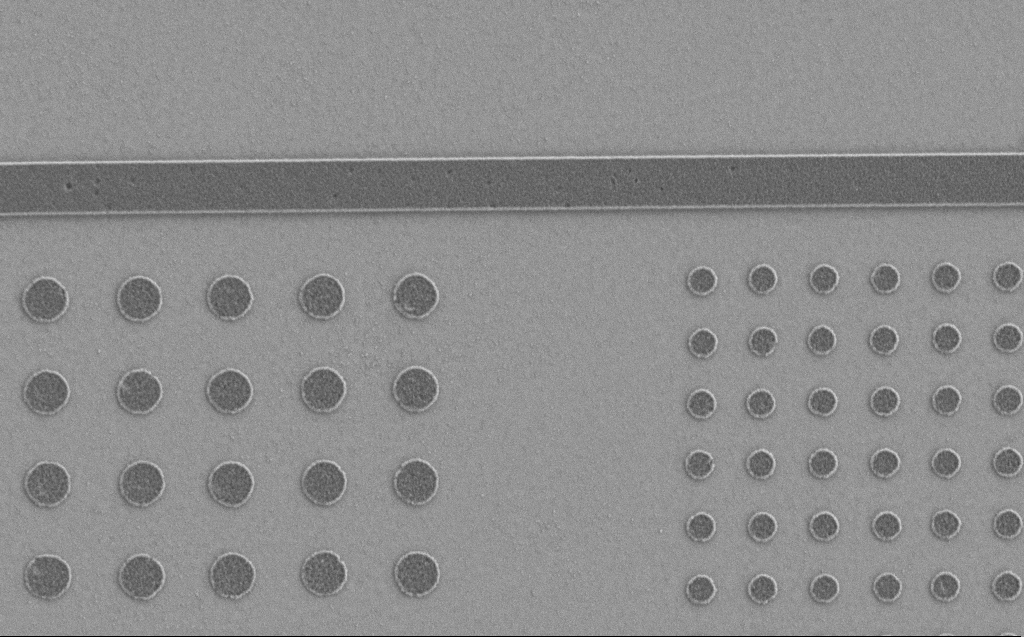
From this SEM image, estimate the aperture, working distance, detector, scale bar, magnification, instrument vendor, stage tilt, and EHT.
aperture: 30 µm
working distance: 5 mm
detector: SE2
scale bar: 2000 nm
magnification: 18.88 K X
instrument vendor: Zeiss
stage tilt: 0°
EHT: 1.2 kV